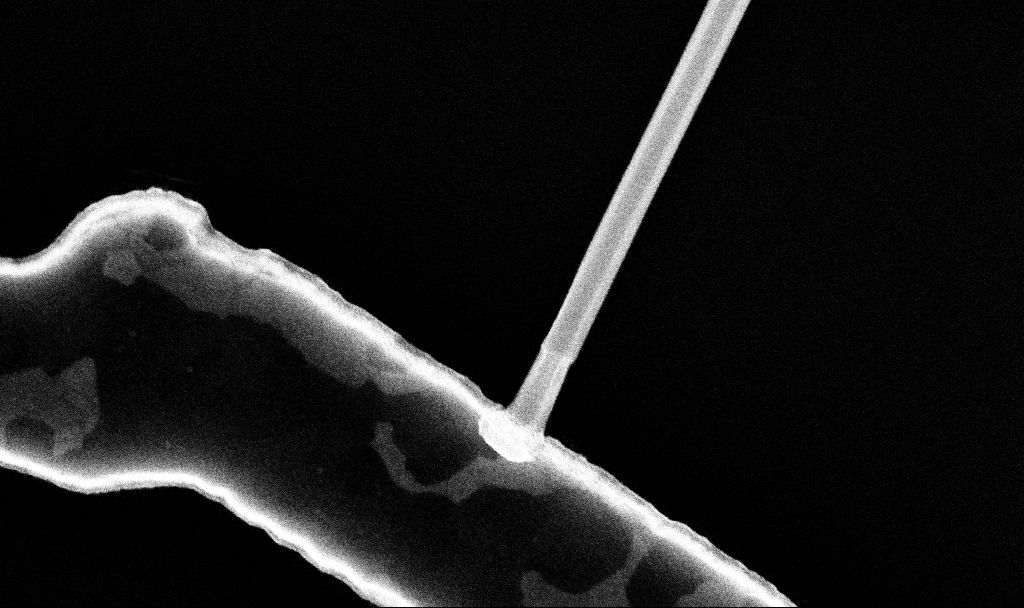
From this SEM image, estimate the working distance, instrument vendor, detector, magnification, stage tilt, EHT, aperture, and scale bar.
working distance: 7.7 mm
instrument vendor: Zeiss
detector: InLens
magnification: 106.15 K X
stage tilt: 0°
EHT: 10 kV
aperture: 30 µm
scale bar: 200 nm